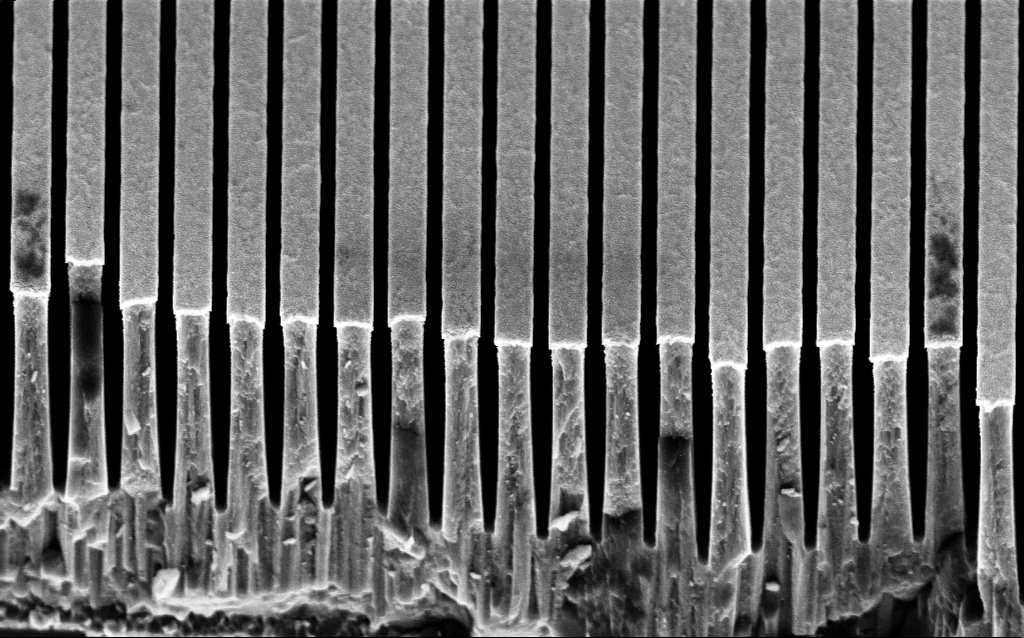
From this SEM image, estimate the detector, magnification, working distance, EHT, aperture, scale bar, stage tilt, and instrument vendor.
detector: InLens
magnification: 39.78 K X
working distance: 6 mm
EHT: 3 kV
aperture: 30 µm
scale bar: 1000 nm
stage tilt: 45°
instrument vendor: Zeiss